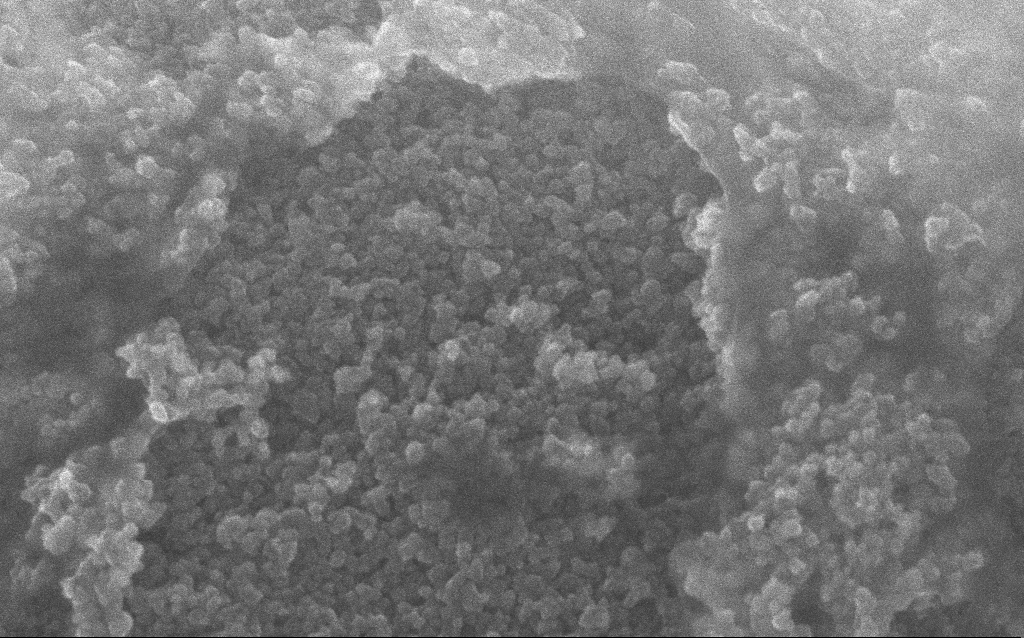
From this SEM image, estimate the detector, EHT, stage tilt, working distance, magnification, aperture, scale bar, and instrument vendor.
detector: InLens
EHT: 10 kV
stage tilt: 0°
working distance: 2.8 mm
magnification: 204.13 K X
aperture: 30 µm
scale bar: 100 nm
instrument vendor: Zeiss